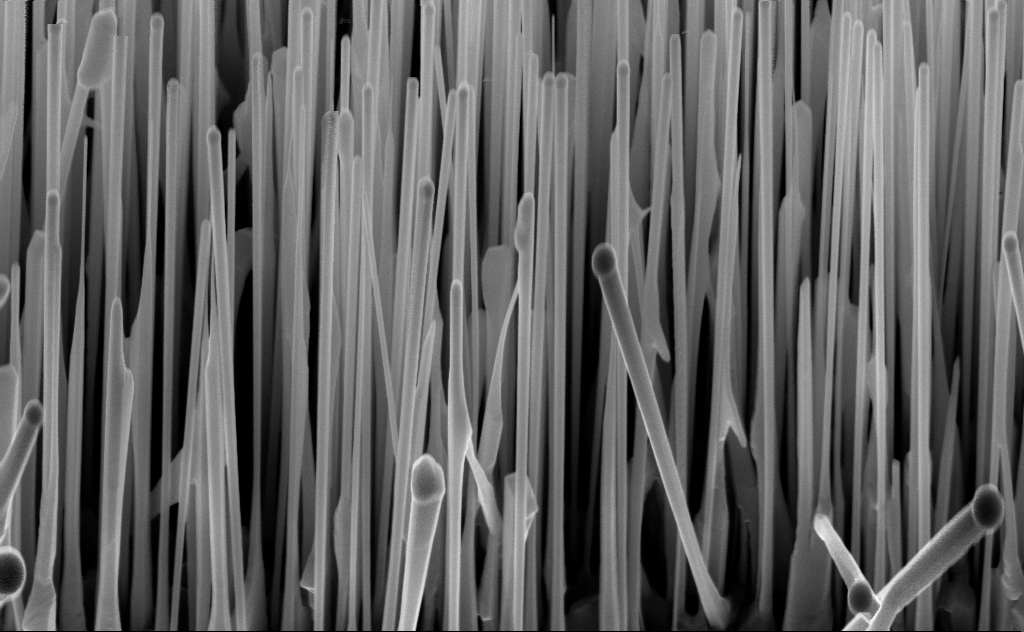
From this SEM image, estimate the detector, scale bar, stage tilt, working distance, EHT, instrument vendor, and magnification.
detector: InLens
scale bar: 1000 nm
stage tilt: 45°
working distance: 6 mm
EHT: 10 kV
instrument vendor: Zeiss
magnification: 40 K X